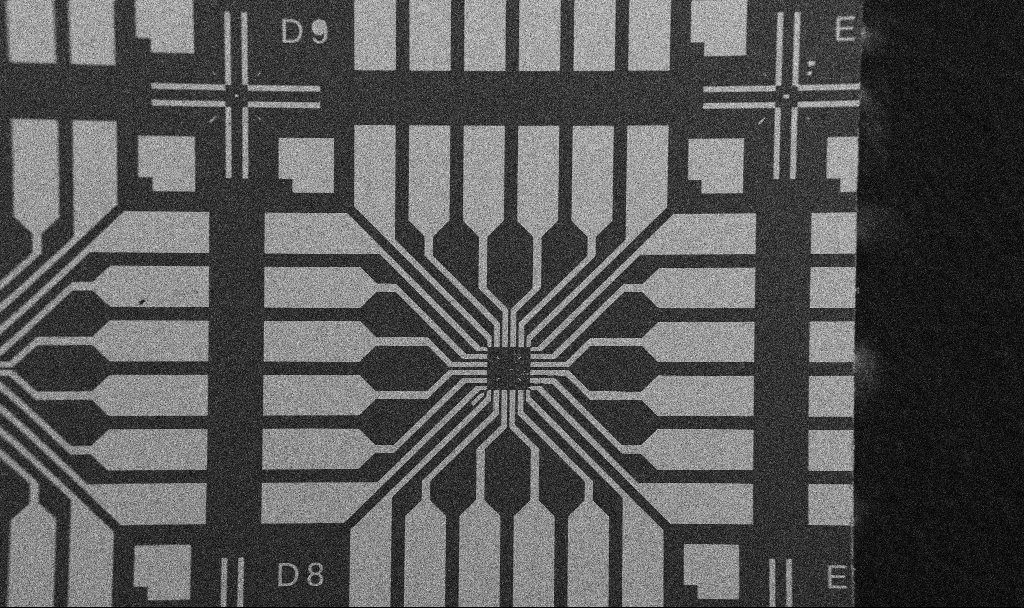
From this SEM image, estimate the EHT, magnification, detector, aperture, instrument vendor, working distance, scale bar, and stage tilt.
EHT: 5 kV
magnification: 0.1 K X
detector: SE2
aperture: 30 µm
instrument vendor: Zeiss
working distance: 10.7 mm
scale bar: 200000 nm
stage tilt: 0°